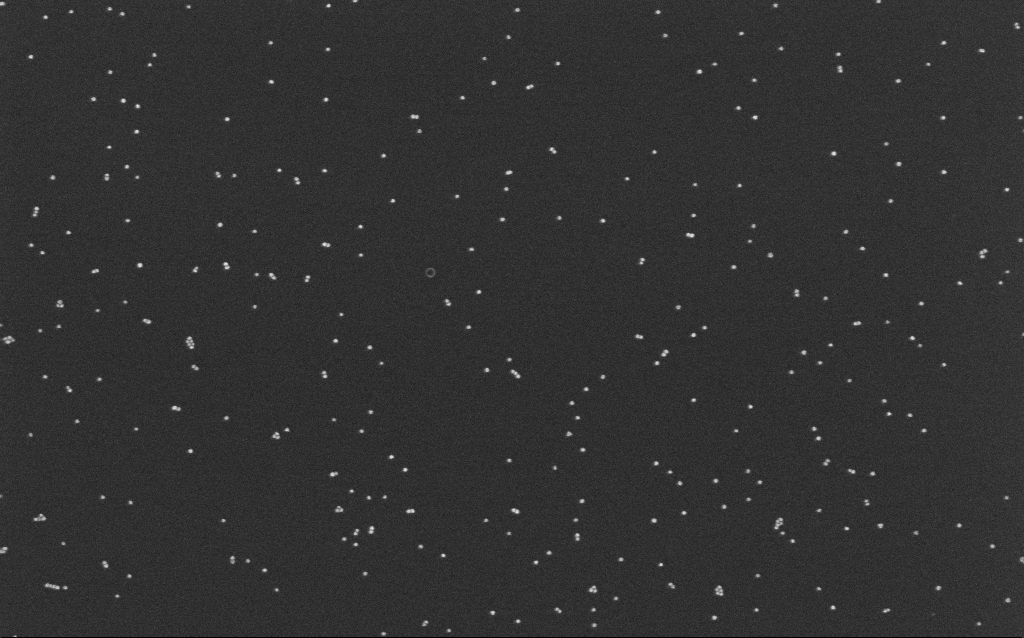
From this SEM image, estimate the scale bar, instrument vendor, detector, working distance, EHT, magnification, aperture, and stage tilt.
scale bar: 200 nm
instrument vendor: Zeiss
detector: InLens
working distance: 6.6 mm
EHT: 10 kV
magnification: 100 K X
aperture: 30 µm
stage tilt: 0°